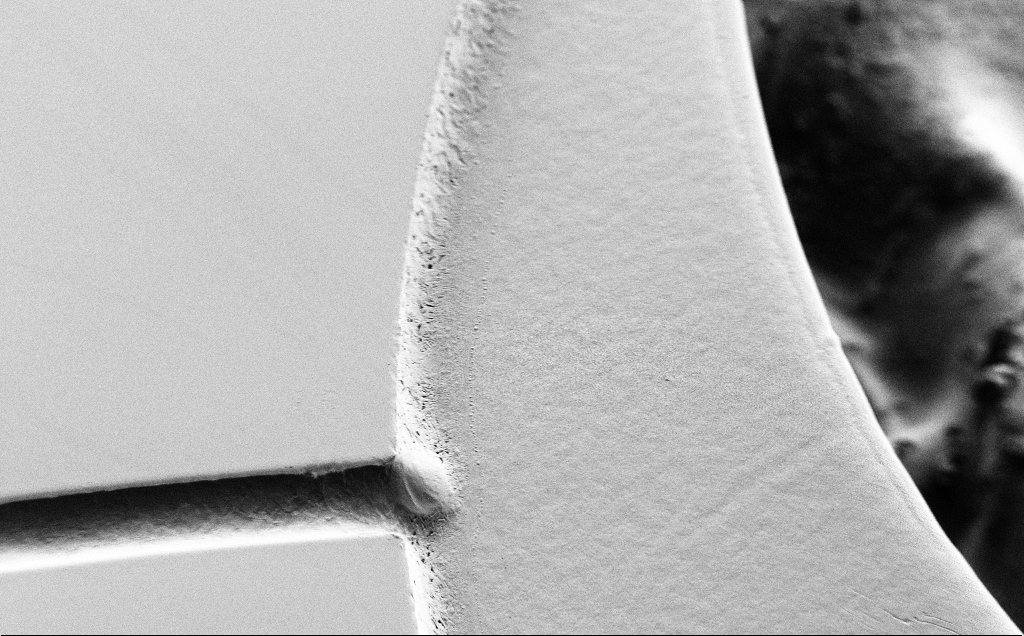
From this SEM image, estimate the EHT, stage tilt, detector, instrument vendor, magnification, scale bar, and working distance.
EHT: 0.8 kV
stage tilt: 45°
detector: SE2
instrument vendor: Zeiss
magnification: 0.509 K X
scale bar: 100000 nm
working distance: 6 mm